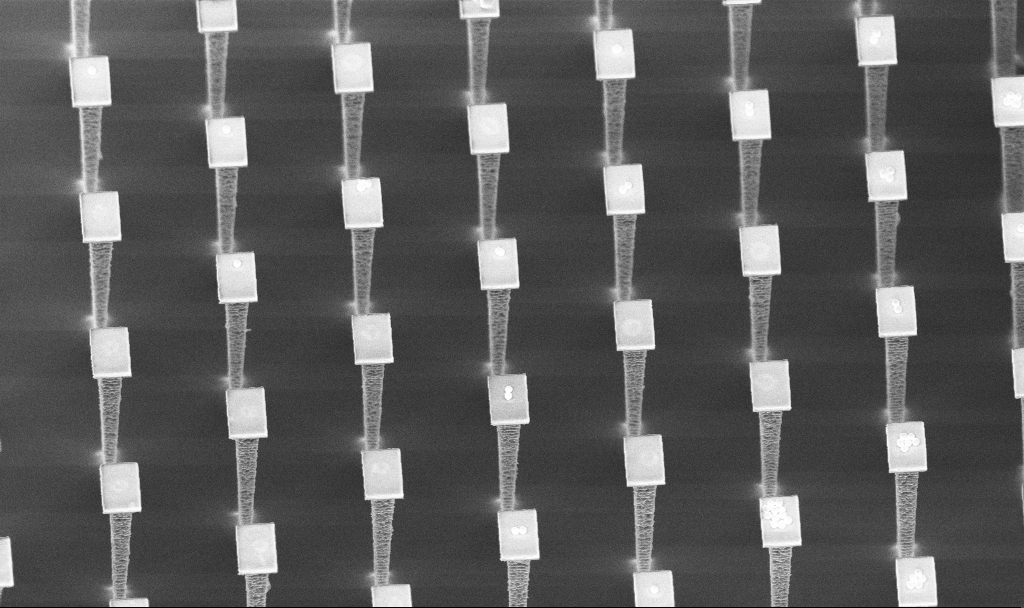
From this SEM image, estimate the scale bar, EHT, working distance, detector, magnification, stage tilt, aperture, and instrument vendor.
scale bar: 10000 nm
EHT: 5 kV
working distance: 4.5 mm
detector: InLens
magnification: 4.82 K X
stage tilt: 30°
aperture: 30 µm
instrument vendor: Zeiss